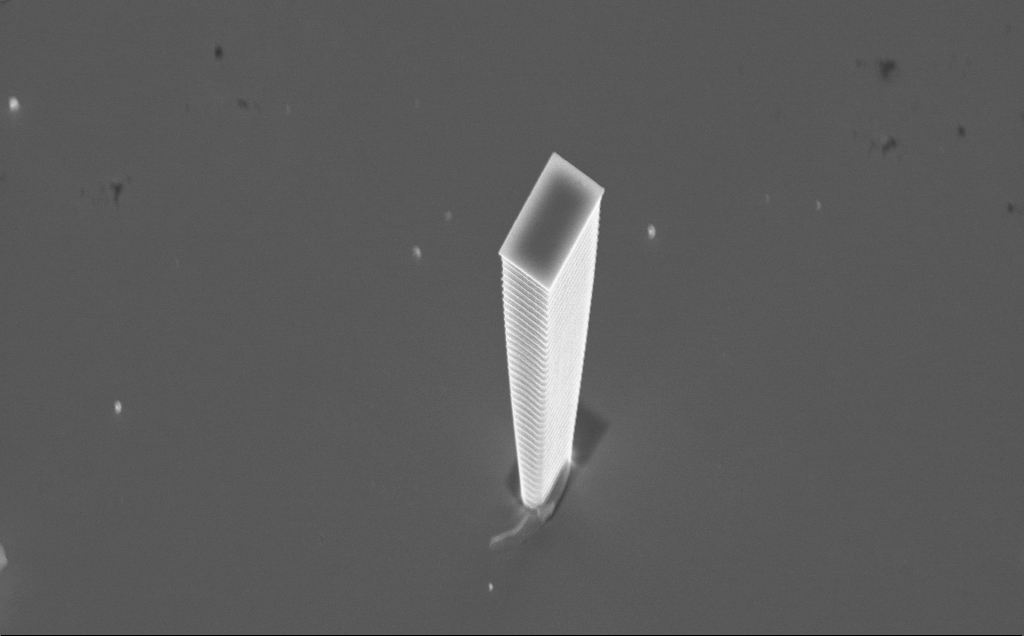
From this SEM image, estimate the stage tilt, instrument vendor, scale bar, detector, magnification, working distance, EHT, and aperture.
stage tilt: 36.9°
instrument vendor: Zeiss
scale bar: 2000 nm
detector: InLens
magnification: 7.79 K X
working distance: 4 mm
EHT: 10 kV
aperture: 30 µm